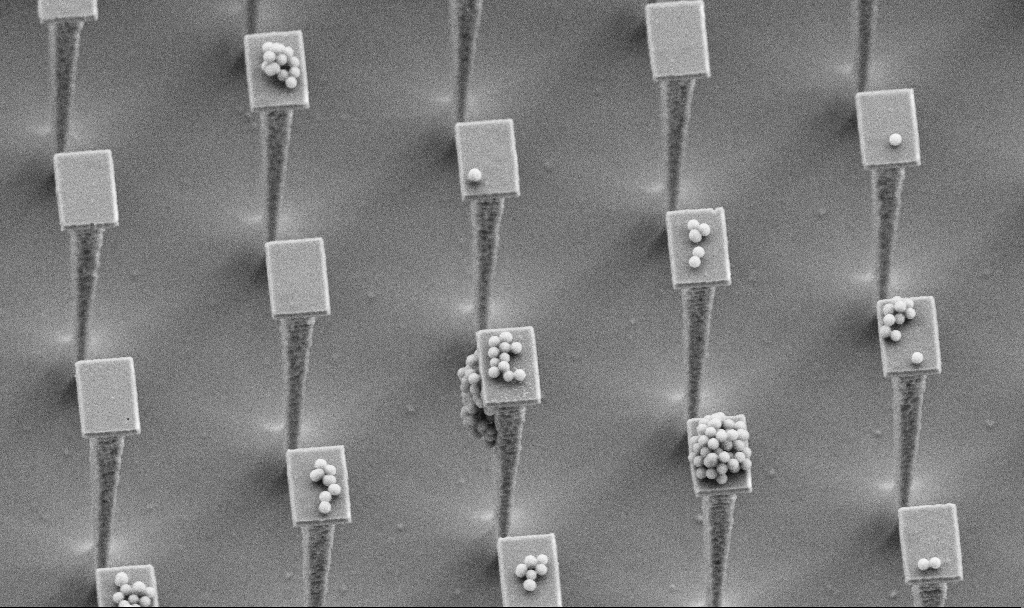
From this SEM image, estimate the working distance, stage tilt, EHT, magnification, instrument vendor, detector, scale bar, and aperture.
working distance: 4.5 mm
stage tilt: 30°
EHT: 5 kV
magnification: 7.35 K X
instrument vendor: Zeiss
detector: SE2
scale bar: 2000 nm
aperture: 30 µm